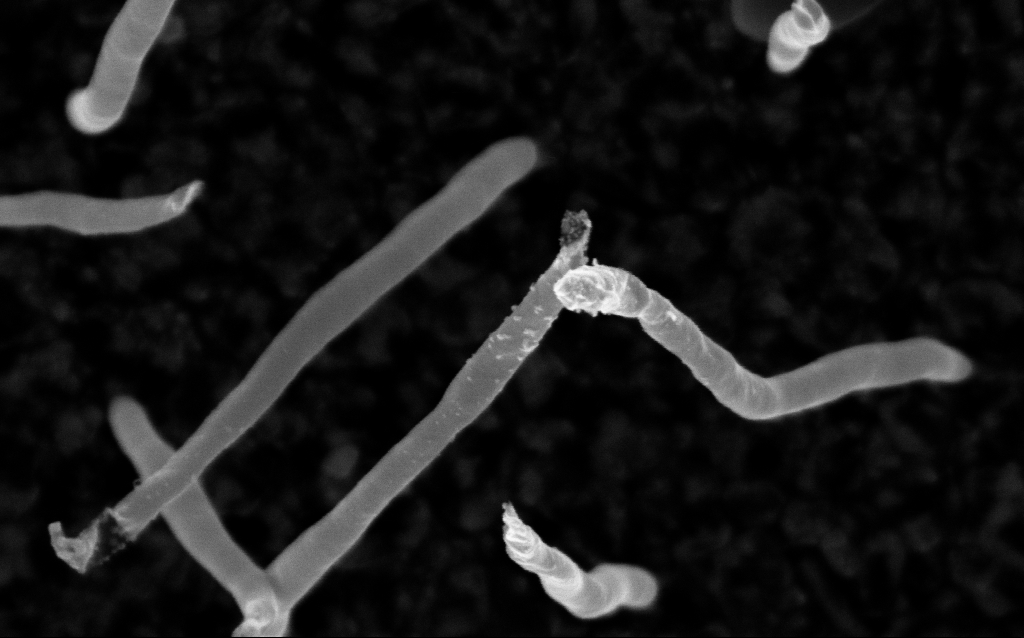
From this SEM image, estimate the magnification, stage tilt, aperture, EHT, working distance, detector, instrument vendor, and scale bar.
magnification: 200 K X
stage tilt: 0°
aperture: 30 µm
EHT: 5 kV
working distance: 2 mm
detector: InLens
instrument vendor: Zeiss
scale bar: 100 nm